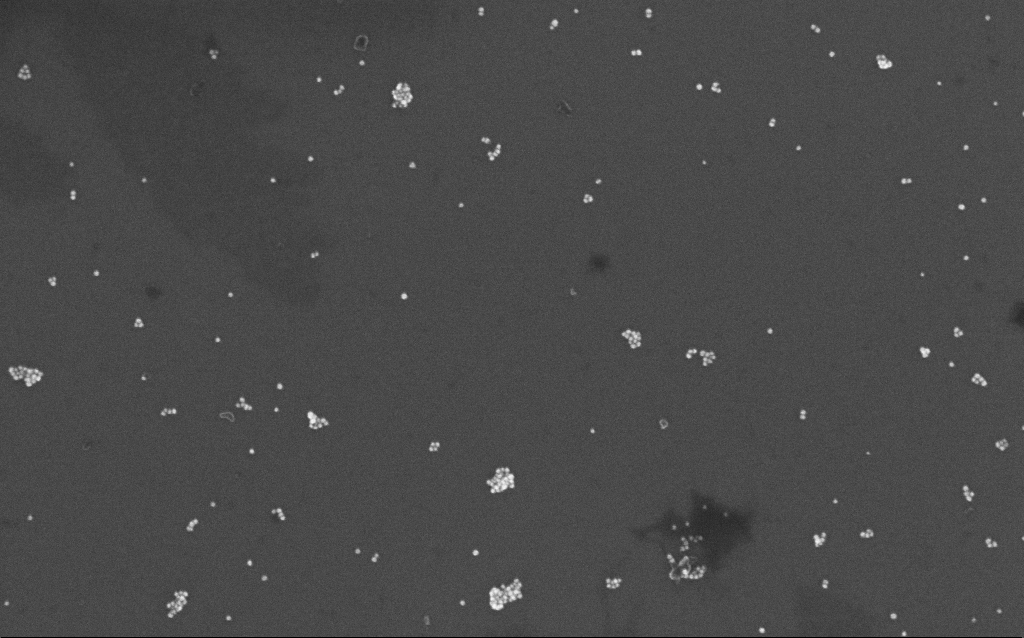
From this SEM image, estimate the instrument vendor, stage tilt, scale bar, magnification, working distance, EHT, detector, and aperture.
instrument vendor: Zeiss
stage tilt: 0°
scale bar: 200 nm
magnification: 96.05 K X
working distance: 7 mm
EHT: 10 kV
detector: InLens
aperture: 30 µm